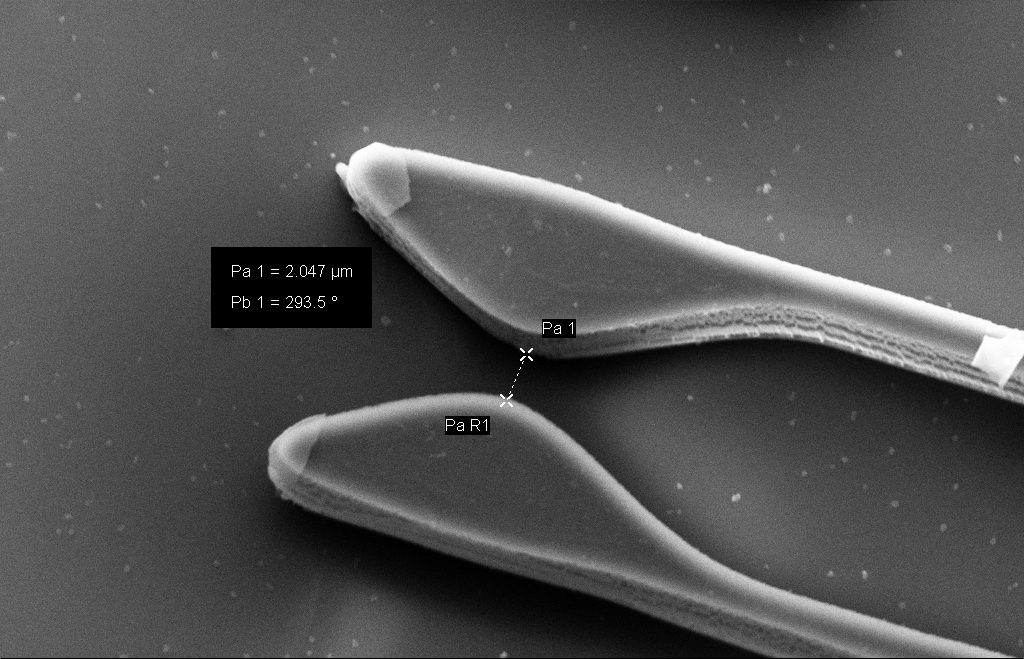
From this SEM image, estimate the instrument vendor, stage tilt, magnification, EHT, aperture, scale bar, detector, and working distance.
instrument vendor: Zeiss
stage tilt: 15.3°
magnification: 9 K X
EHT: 10 kV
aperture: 30 µm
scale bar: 2000 nm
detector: SE2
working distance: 11 mm